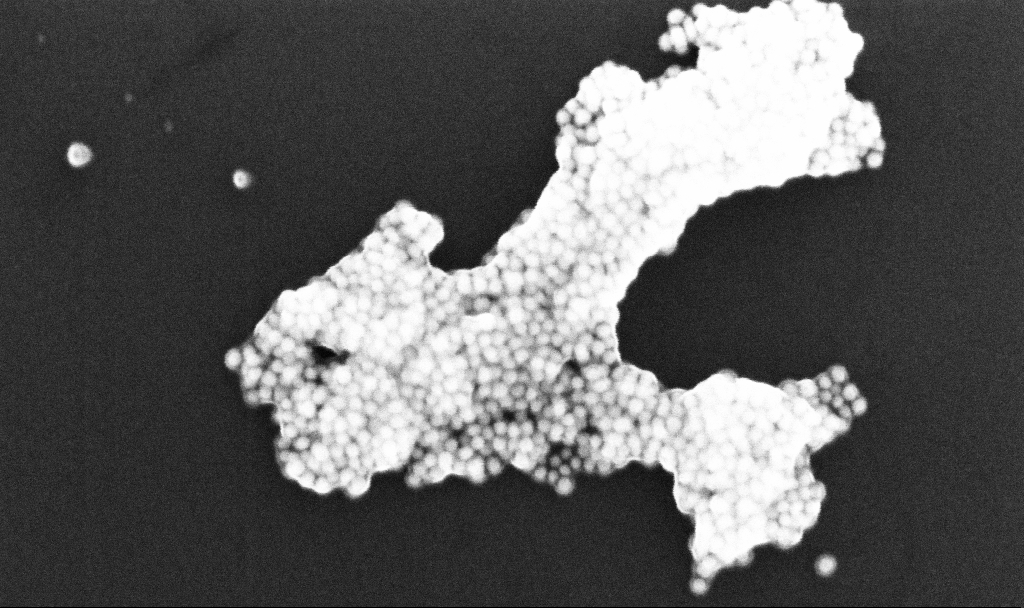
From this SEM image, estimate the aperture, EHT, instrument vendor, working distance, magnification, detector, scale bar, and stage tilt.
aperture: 30 µm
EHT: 10 kV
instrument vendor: Zeiss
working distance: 3.2 mm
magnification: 249.57 K X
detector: InLens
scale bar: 100 nm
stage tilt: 0°